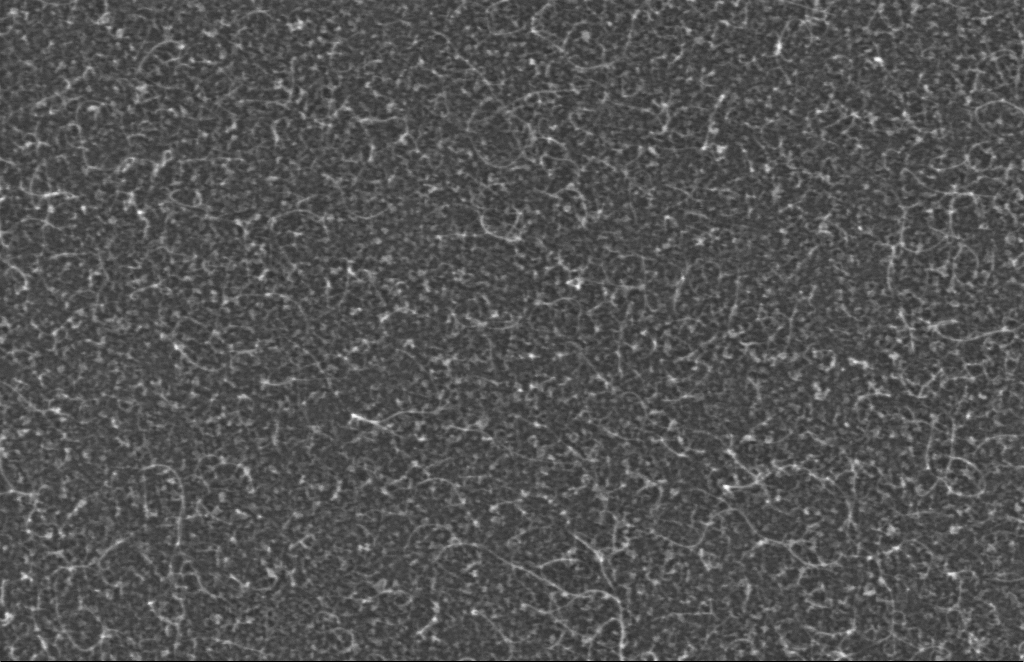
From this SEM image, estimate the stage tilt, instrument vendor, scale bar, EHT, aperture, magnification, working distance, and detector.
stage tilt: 0°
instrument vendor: Zeiss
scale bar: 100 nm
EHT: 5 kV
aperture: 30 µm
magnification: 223.63 K X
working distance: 6 mm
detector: InLens